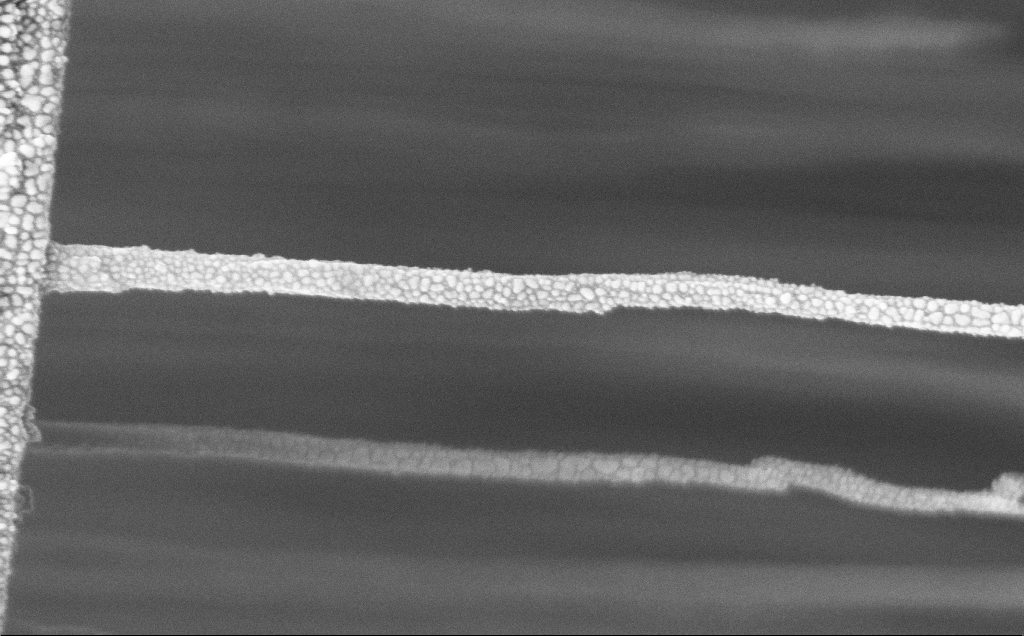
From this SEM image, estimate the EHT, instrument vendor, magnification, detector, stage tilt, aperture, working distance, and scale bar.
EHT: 5 kV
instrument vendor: Zeiss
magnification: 89.56 K X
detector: InLens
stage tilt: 0°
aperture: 30 µm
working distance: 12 mm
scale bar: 200 nm